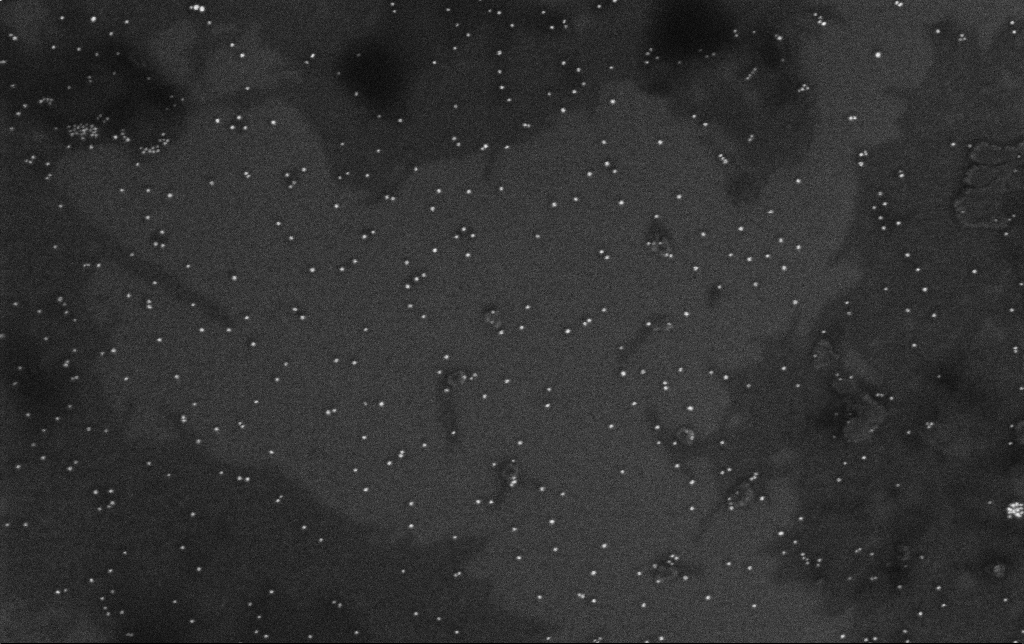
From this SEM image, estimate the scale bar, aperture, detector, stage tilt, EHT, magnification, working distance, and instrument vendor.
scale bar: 200 nm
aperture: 30 µm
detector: InLens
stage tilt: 0°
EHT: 10 kV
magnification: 100 K X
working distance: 3.2 mm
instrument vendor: Zeiss